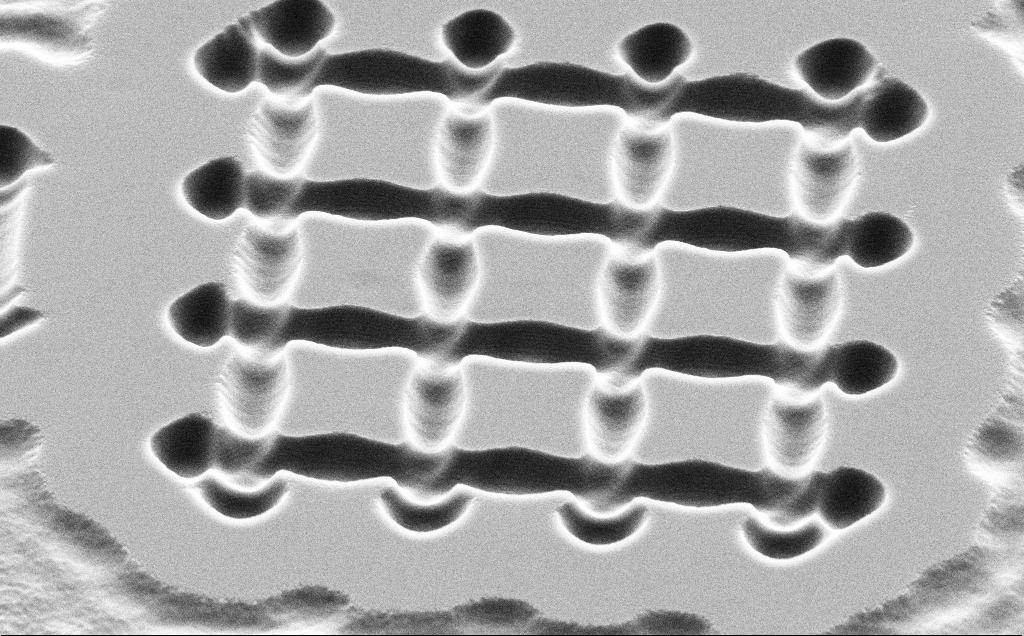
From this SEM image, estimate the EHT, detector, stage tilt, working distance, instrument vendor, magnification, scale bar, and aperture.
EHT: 5 kV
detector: SE2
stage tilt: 45°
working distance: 11 mm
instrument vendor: Zeiss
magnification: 10.3 K X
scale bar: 2000 nm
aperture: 30 µm